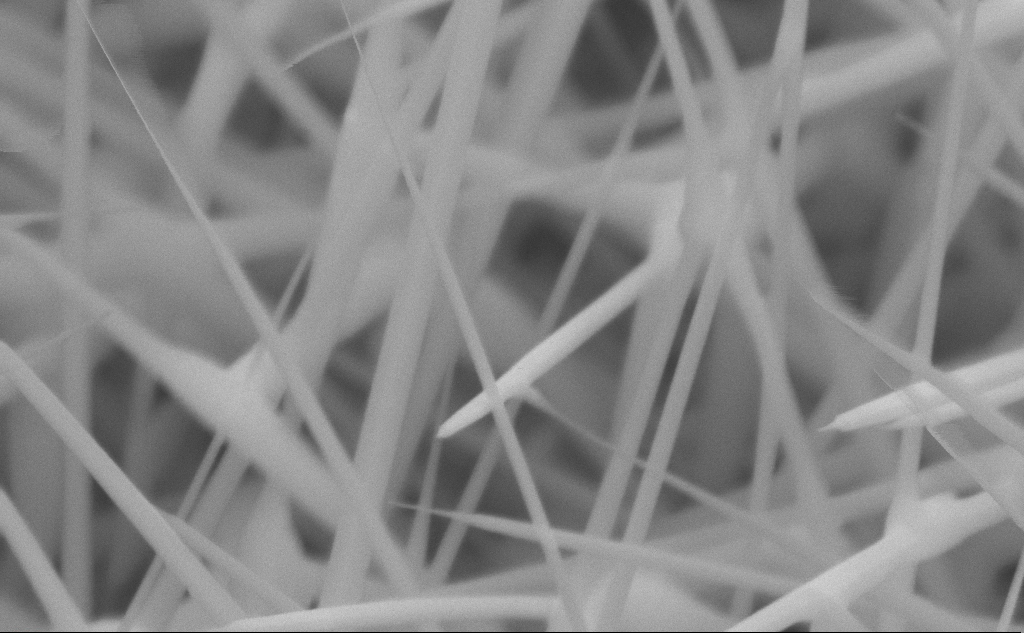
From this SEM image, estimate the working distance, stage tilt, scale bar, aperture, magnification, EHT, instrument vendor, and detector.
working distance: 5 mm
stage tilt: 45°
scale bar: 200 nm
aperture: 30 µm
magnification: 150 K X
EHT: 10 kV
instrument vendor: Zeiss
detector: SE2